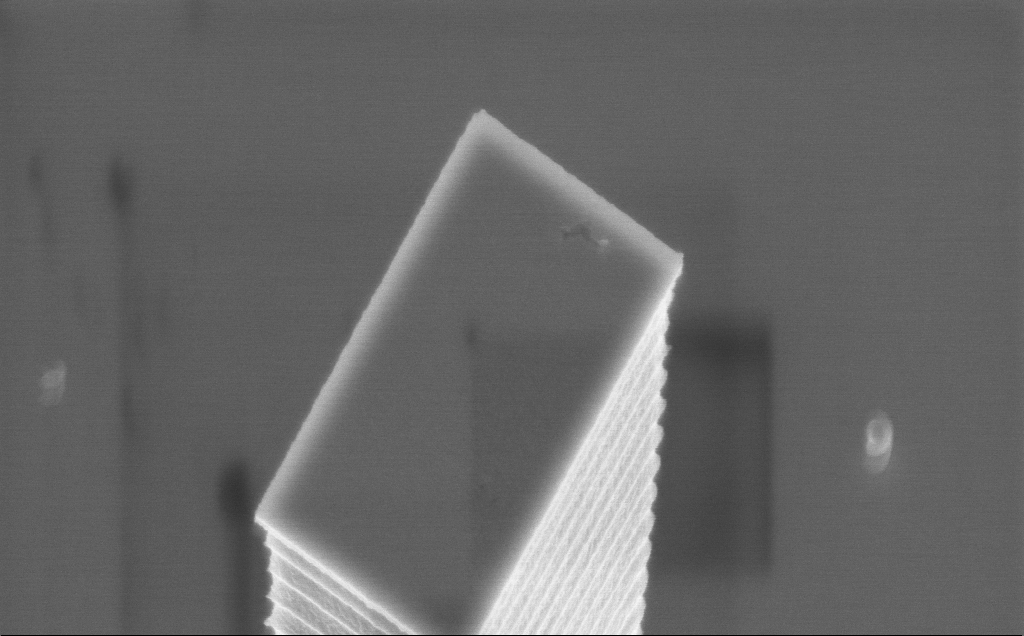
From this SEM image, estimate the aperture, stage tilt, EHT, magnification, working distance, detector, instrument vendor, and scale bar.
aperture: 30 µm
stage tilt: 36.9°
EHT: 5 kV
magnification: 31.48 K X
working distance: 4 mm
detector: InLens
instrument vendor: Zeiss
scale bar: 1000 nm